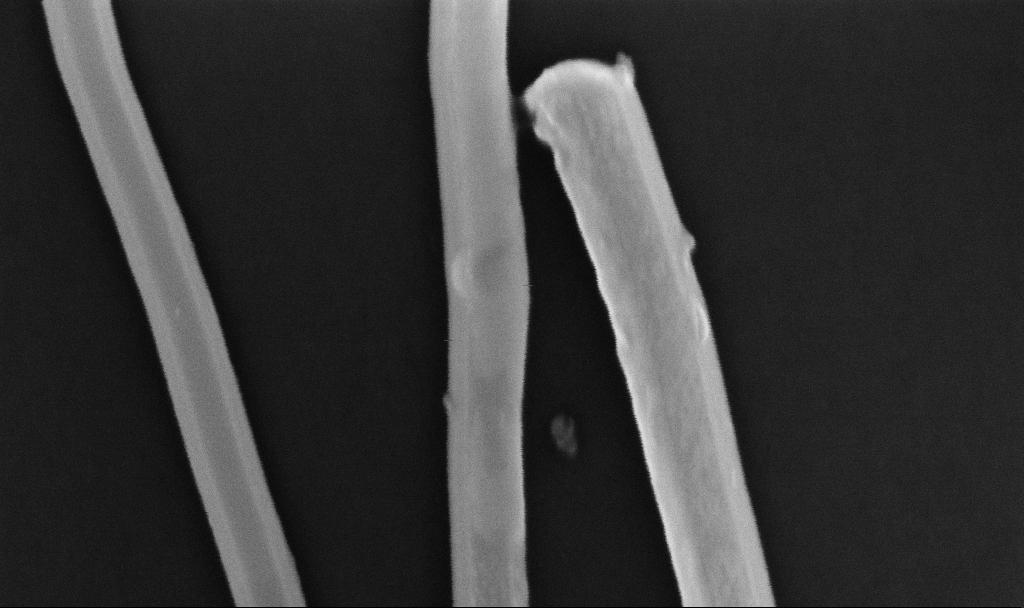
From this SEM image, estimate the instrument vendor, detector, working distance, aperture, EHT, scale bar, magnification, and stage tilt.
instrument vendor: Zeiss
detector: InLens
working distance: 6.7 mm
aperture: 30 µm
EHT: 10 kV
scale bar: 200 nm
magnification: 241.15 K X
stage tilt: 0°